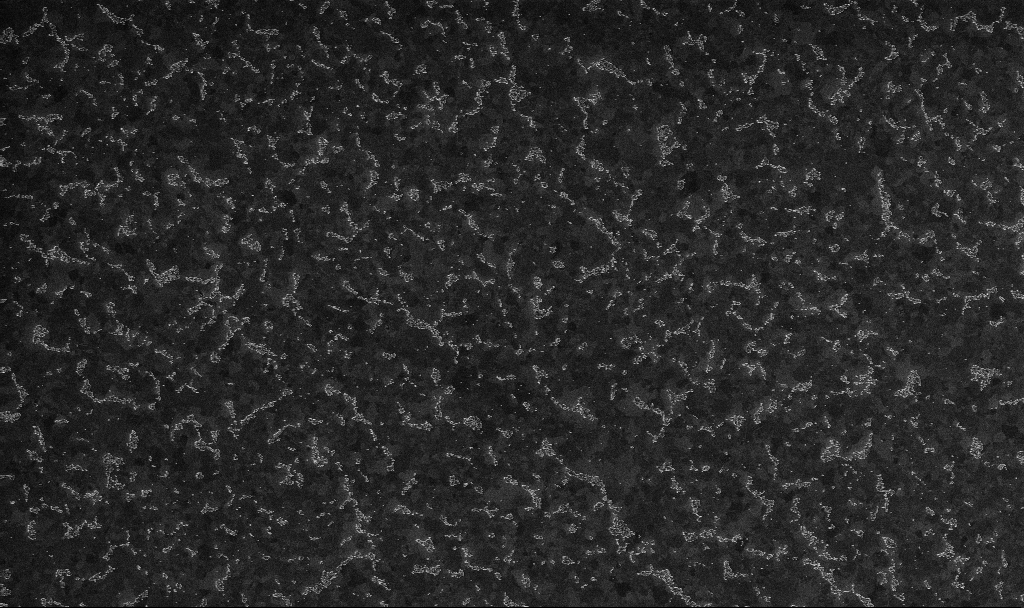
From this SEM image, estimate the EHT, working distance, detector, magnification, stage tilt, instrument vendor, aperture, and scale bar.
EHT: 10 kV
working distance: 3.4 mm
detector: InLens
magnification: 20 K X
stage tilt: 0°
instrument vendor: Zeiss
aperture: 30 µm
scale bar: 1000 nm